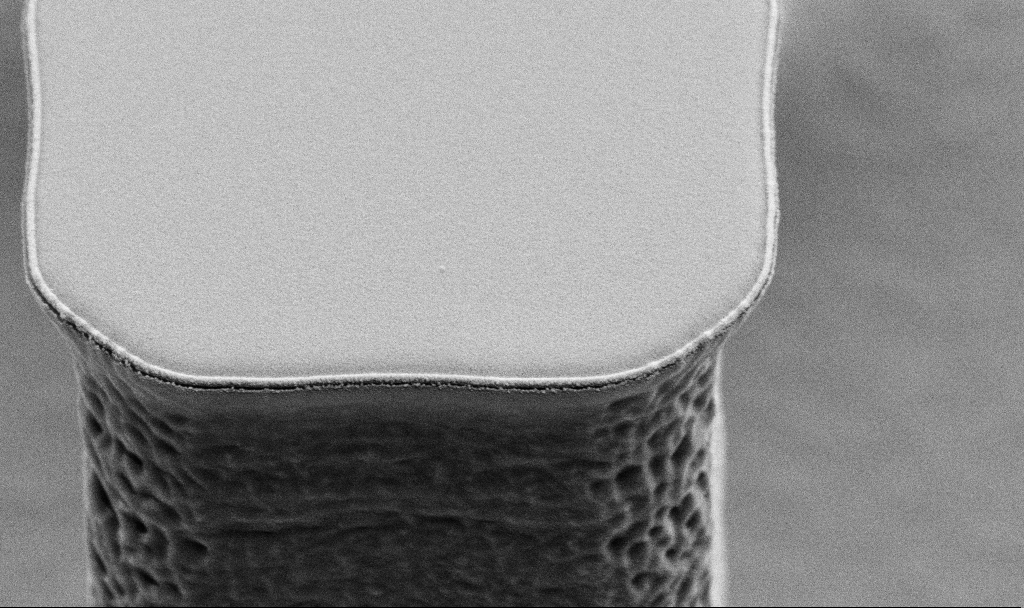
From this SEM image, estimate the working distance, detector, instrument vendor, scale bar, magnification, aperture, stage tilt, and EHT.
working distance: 8.2 mm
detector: SE2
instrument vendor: Zeiss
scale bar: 1000 nm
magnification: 28.62 K X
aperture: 30 µm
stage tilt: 45°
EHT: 5 kV